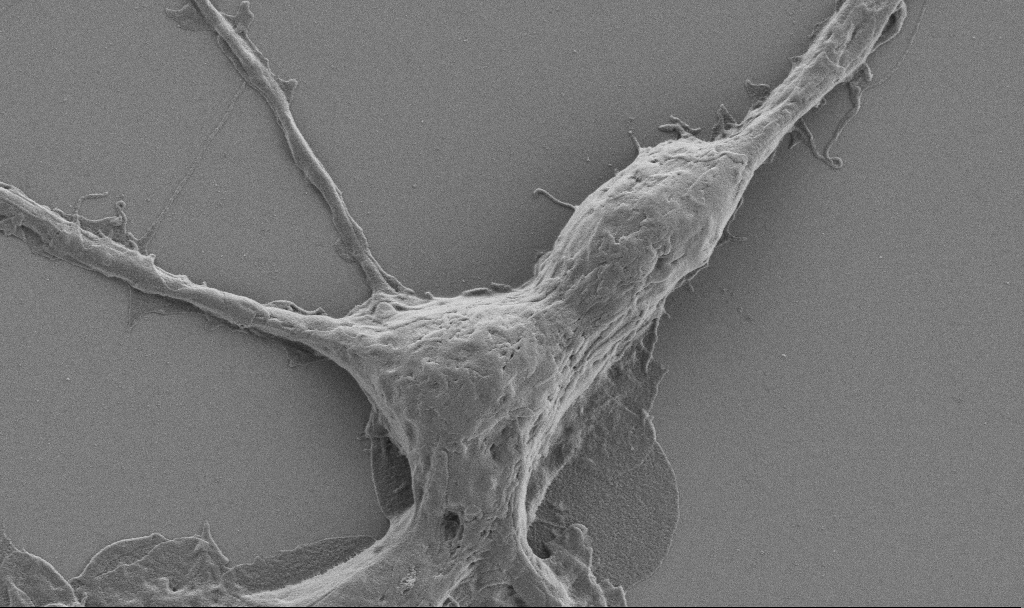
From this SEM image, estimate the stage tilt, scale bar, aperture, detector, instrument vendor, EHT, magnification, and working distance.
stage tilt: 0°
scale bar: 2000 nm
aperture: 30 µm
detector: SE2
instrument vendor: Zeiss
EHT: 0.9 kV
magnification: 10 K X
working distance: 6.9 mm